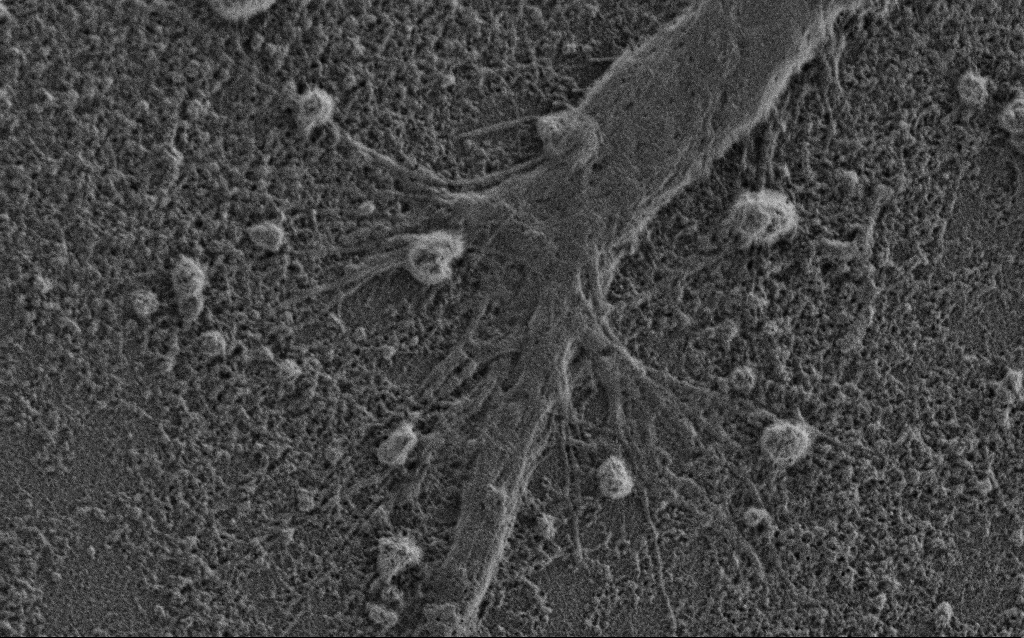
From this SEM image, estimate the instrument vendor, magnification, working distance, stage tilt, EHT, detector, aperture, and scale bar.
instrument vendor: Zeiss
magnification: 10 K X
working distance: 3.4 mm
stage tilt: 0°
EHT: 0.9 kV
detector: SE2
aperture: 30 µm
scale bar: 2000 nm